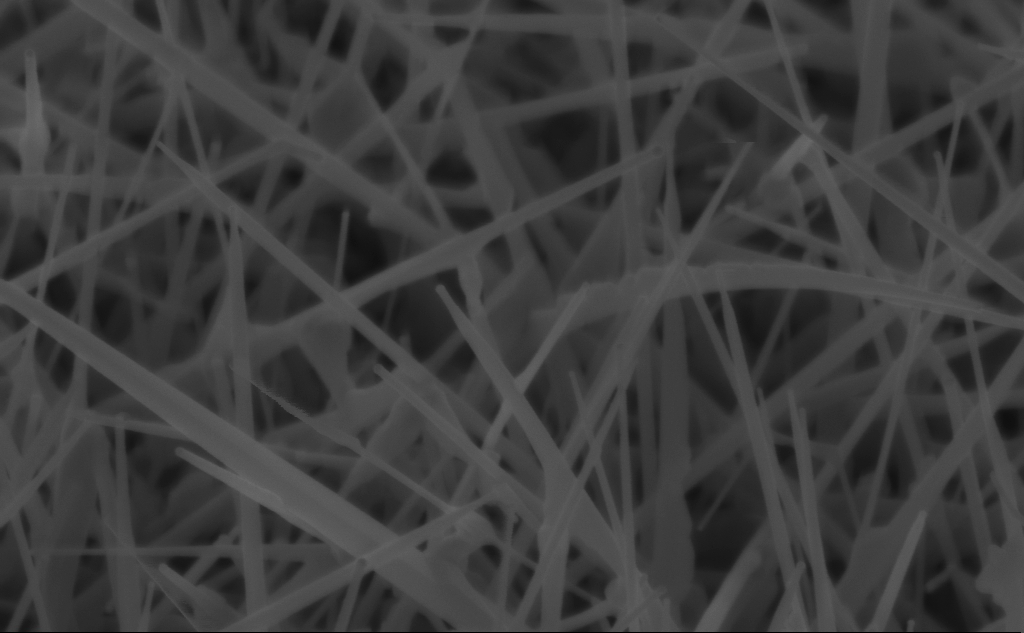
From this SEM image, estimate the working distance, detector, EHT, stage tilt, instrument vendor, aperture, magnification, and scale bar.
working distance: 4 mm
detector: InLens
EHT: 5 kV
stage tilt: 45°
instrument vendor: Zeiss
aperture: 30 µm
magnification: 95.14 K X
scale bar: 200 nm